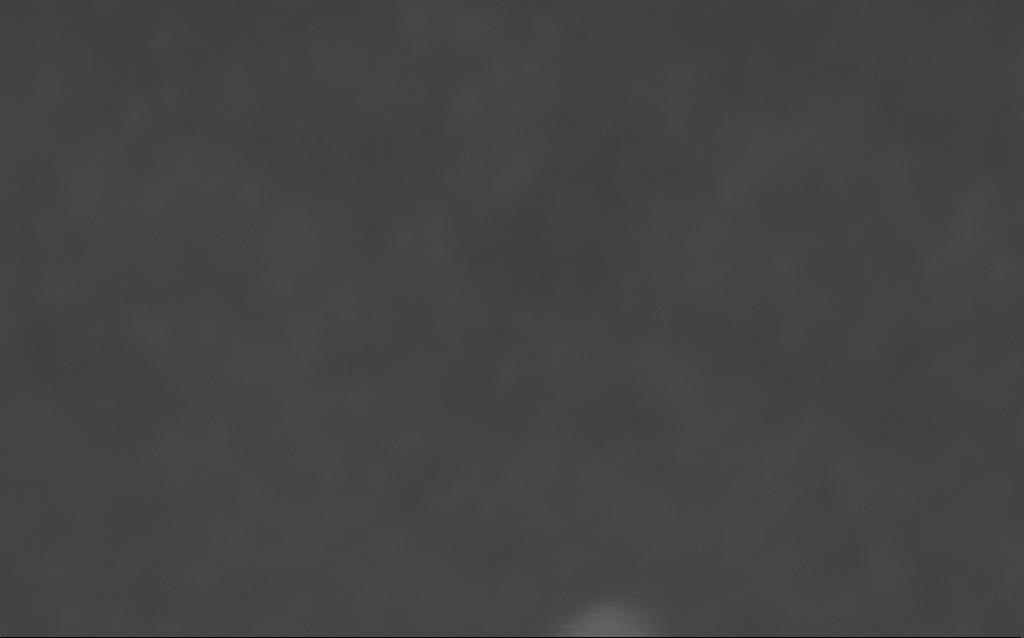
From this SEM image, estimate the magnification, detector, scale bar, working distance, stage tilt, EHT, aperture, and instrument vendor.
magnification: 3204.55 K X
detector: InLens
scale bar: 20 nm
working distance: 2 mm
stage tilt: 0°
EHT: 10 kV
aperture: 30 µm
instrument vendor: Zeiss